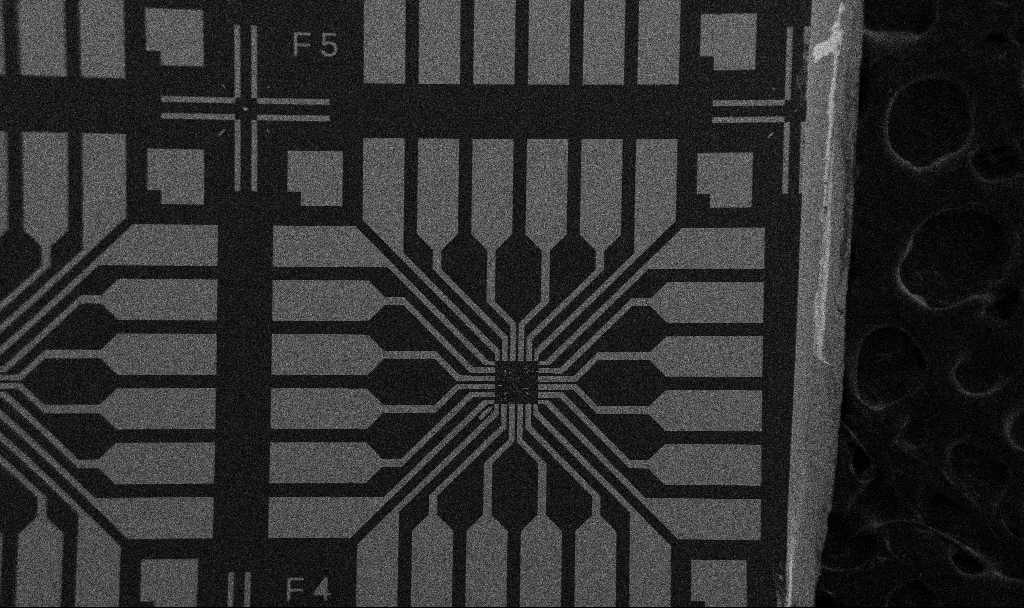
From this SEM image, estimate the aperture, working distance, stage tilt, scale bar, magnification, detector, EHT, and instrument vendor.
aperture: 30 µm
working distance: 10.7 mm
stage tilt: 0°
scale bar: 200000 nm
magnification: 0.1 K X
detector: SE2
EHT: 5 kV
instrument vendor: Zeiss